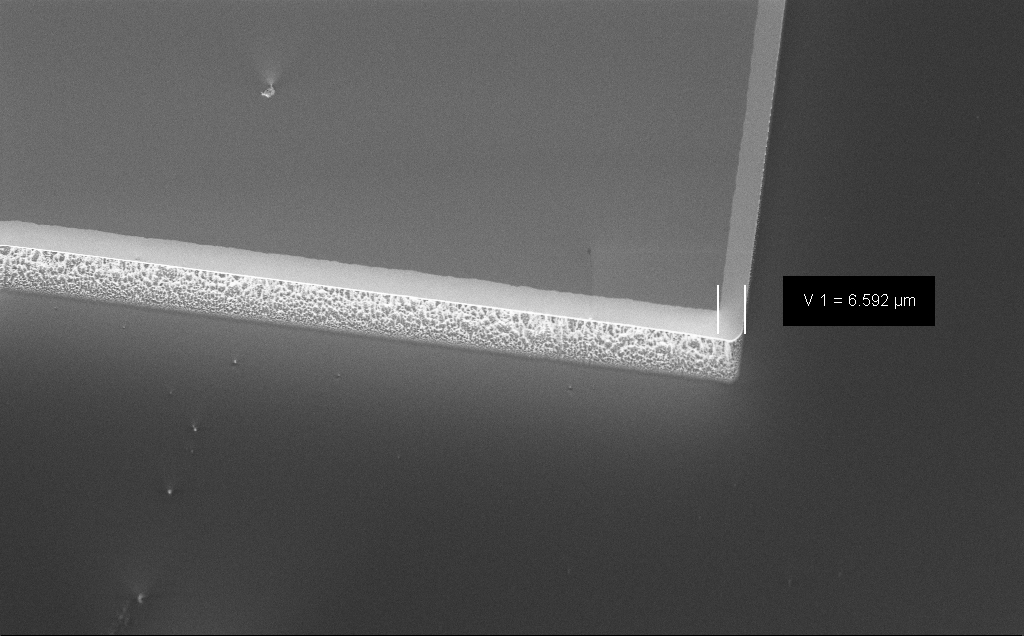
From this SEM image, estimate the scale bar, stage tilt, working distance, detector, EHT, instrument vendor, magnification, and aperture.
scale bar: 20000 nm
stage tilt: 45°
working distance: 7 mm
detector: InLens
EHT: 5 kV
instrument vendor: Zeiss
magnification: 1.5 K X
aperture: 30 µm